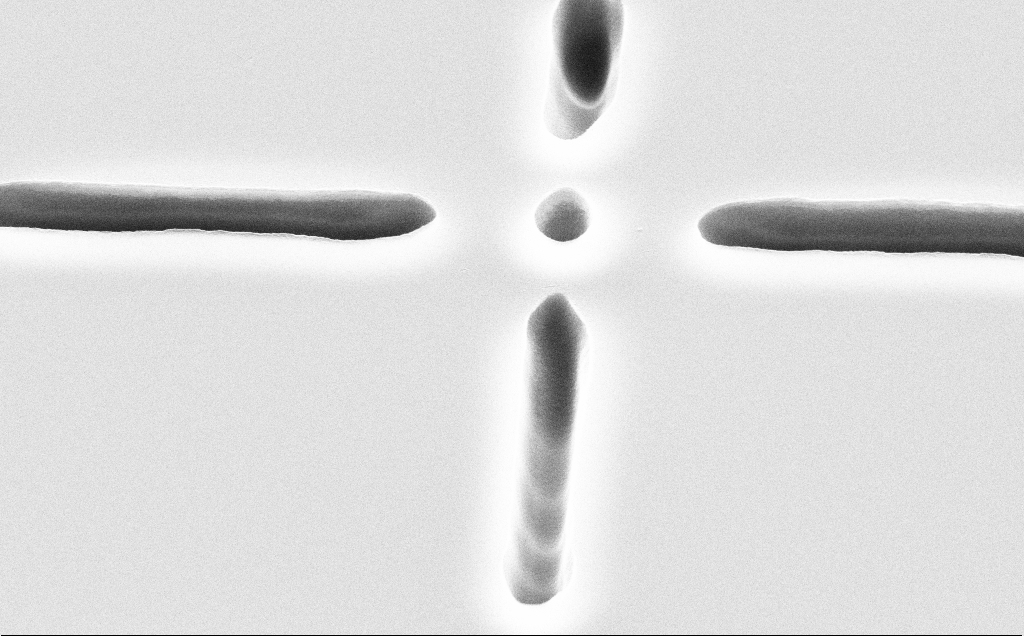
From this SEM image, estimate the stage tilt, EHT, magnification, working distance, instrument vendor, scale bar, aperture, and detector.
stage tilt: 45°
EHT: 10 kV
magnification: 13 K X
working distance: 13 mm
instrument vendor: Zeiss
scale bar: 2000 nm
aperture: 30 µm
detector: SE2